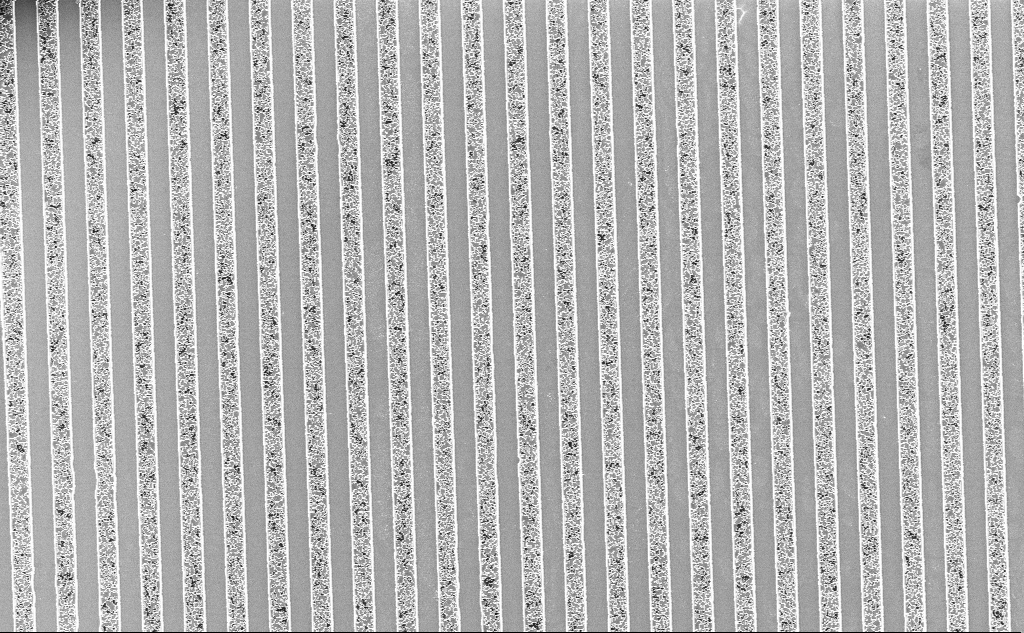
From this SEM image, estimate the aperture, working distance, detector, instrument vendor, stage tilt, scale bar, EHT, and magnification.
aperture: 30 µm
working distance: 7.9 mm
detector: InLens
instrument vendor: Zeiss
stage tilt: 0°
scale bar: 10000 nm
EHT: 3 kV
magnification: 3.87 K X